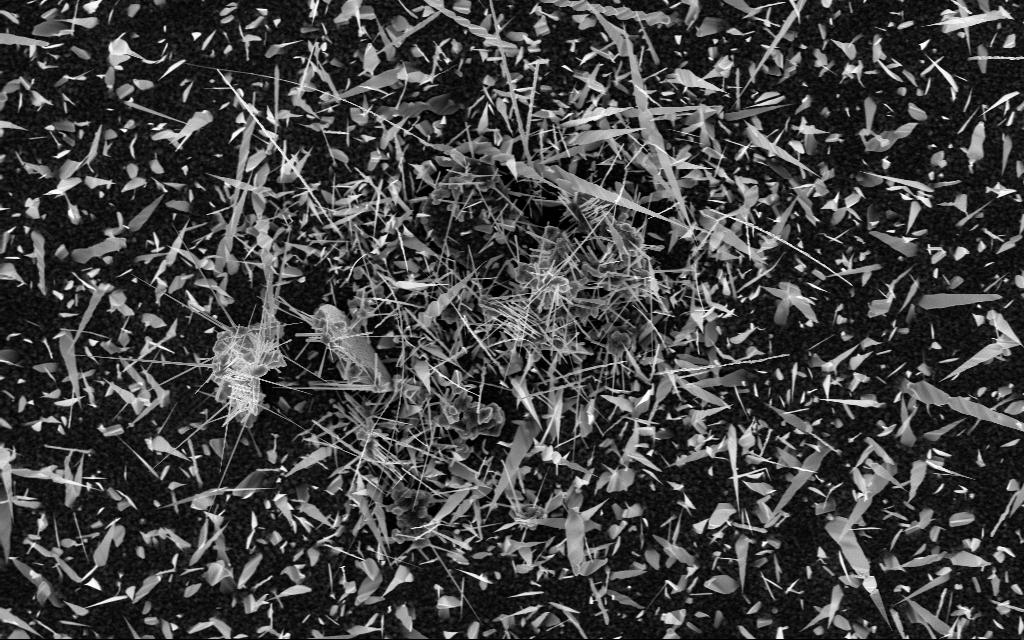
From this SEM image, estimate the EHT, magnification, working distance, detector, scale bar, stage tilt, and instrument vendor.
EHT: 10 kV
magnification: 5 K X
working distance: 6 mm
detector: InLens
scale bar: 10000 nm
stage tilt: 0°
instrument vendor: Zeiss